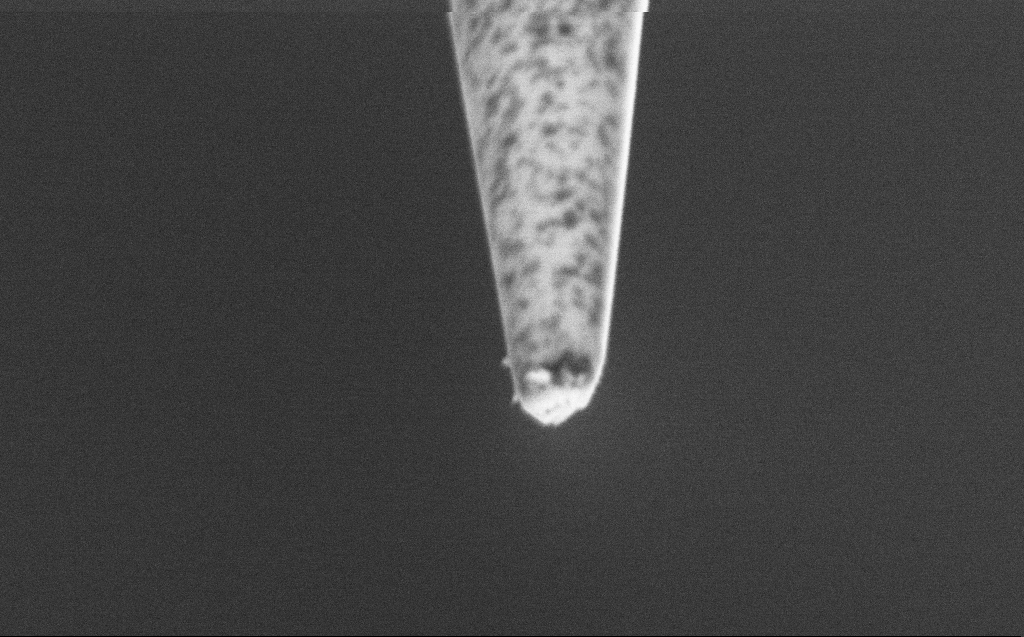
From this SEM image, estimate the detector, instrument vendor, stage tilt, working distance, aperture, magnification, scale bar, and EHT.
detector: SE2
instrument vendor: Zeiss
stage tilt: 45°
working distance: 6 mm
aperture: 30 µm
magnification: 100 K X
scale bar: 200 nm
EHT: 2 kV